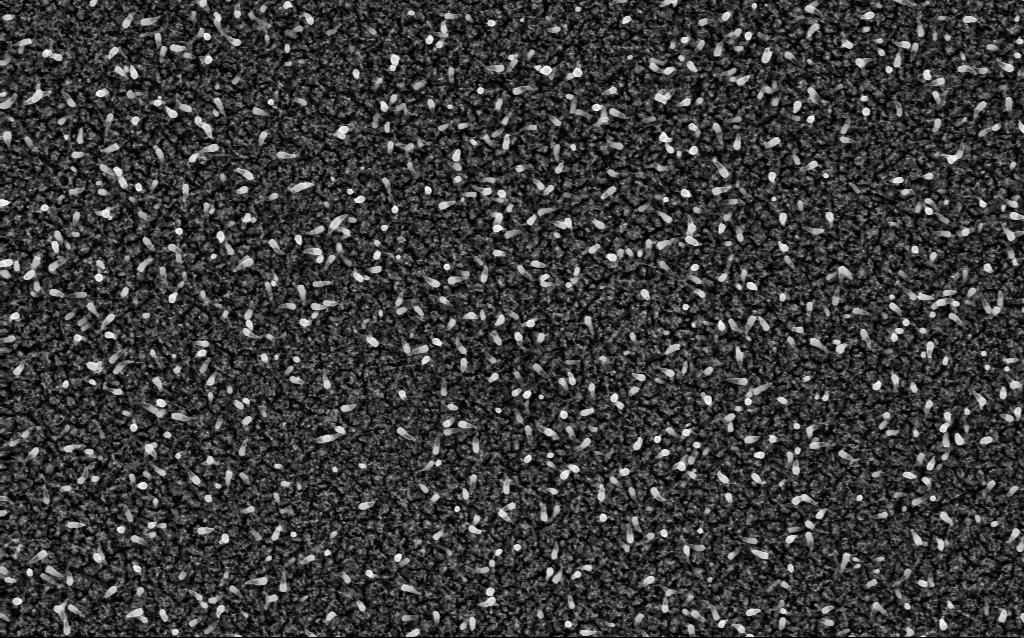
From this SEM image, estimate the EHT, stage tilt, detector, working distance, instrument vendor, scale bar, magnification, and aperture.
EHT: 5 kV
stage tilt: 0°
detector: InLens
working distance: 1.8 mm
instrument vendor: Zeiss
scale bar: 1000 nm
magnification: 50 K X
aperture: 30 µm